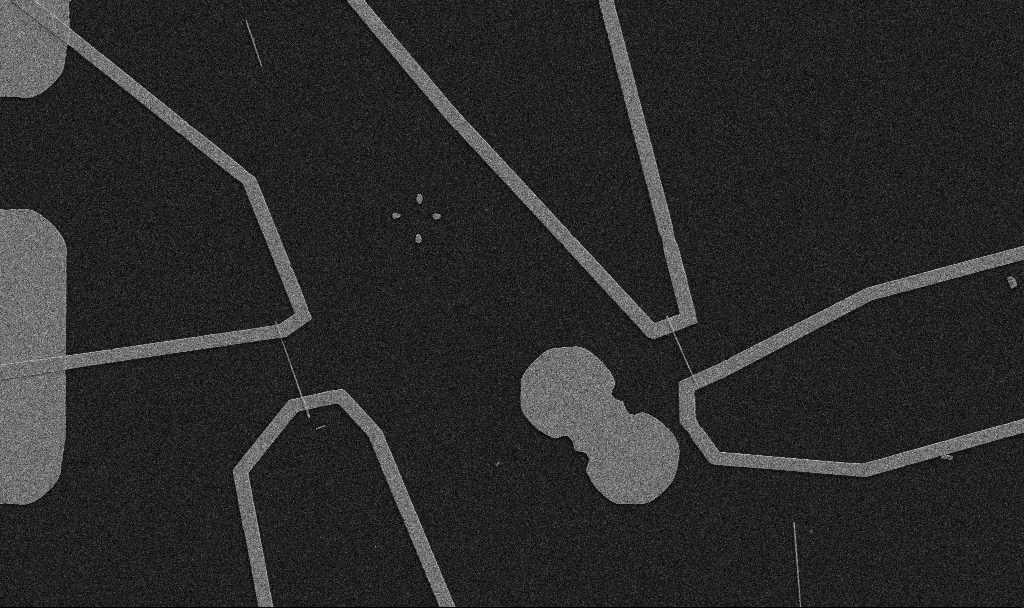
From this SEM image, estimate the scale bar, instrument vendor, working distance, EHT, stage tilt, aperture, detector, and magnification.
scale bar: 10000 nm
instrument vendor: Zeiss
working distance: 10.7 mm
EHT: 5 kV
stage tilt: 0°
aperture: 30 µm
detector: SE2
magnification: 5 K X